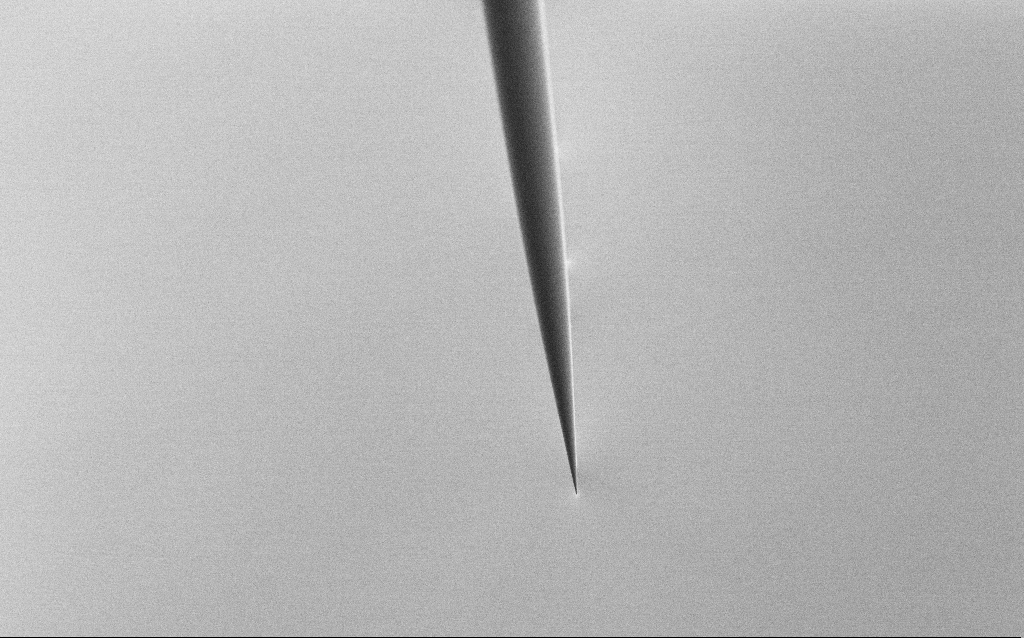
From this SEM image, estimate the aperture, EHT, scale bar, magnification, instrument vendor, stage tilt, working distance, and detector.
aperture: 30 µm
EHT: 2.5 kV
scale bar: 20000 nm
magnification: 1 K X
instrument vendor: Zeiss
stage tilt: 45°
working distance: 5 mm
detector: SE2